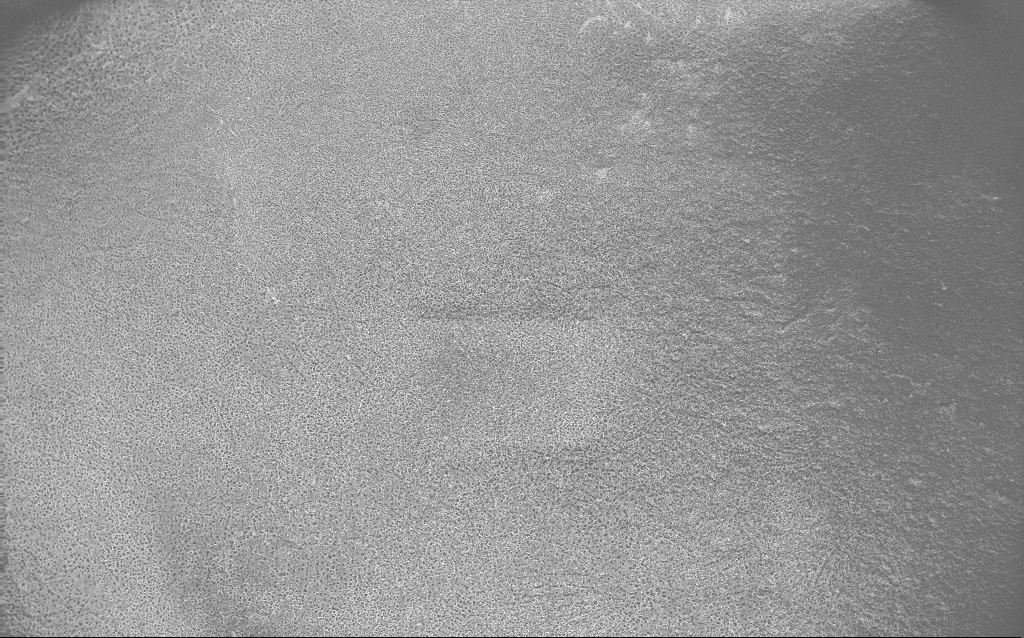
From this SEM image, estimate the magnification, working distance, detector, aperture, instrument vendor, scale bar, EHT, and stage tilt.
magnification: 0.125 K X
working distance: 2.4 mm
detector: InLens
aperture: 30 µm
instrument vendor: Zeiss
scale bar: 100000 nm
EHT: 10 kV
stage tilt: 0°